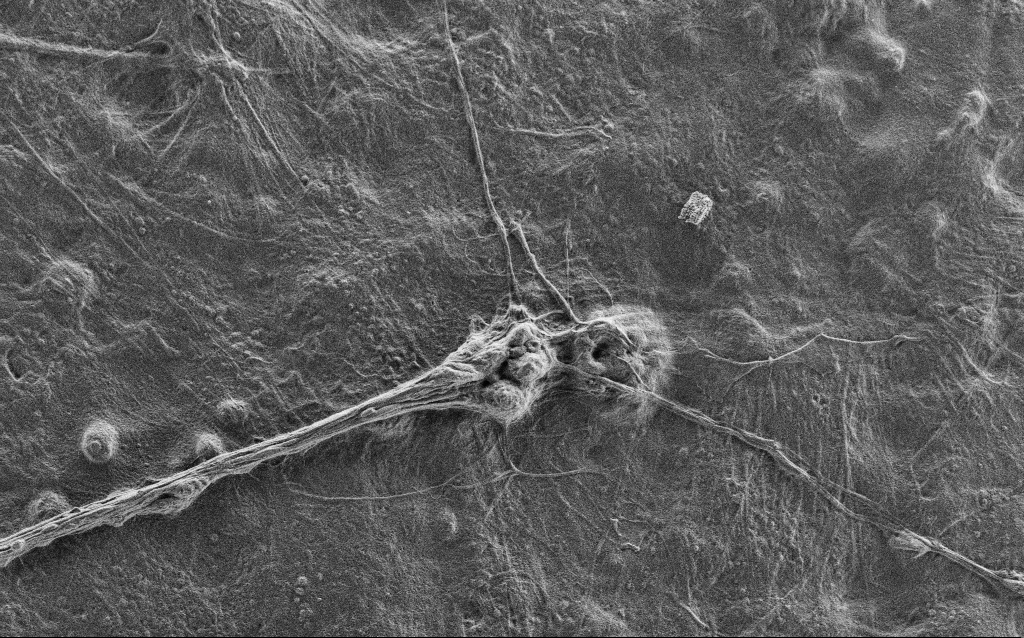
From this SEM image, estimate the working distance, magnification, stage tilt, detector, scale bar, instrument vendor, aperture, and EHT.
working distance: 4 mm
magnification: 5 K X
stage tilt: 0°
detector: SE2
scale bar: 10000 nm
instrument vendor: Zeiss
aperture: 30 µm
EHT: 0.9 kV